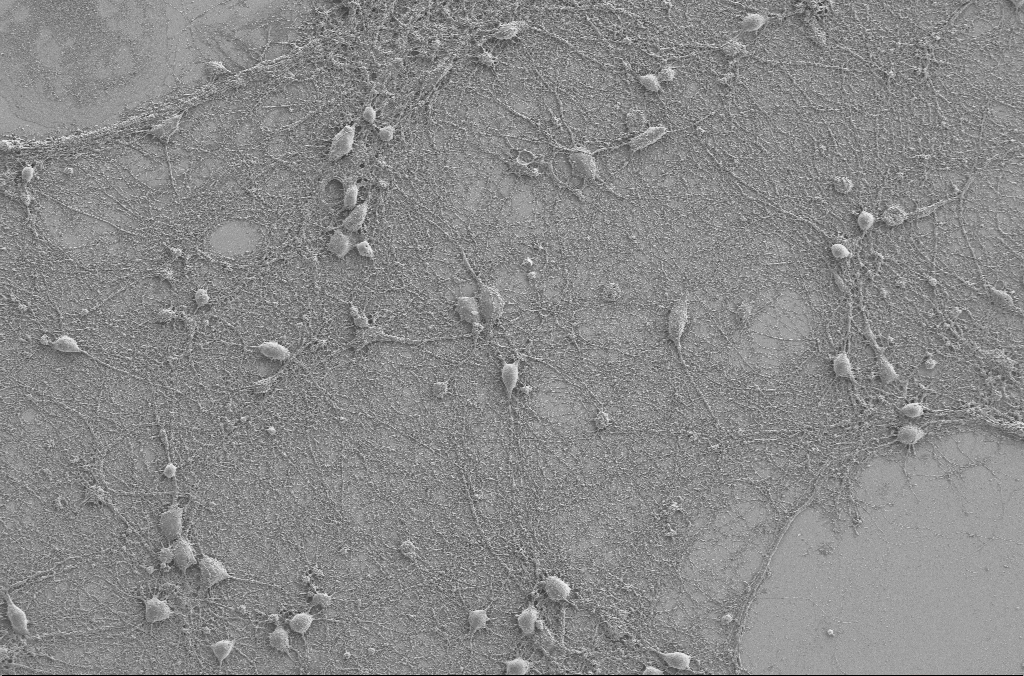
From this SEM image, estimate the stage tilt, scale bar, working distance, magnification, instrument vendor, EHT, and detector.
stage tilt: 0°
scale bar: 20000 nm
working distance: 4 mm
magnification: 1 K X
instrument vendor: Zeiss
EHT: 2 kV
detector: SE2